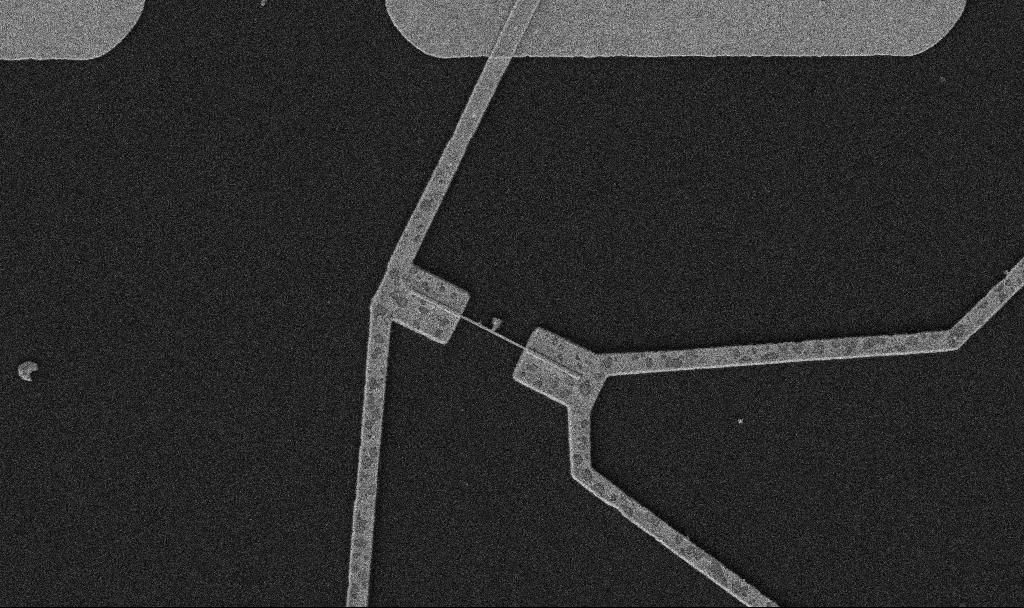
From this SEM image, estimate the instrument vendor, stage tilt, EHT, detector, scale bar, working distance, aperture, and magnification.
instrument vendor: Zeiss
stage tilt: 0°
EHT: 5 kV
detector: SE2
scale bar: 2000 nm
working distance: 10.7 mm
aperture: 30 µm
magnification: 10 K X